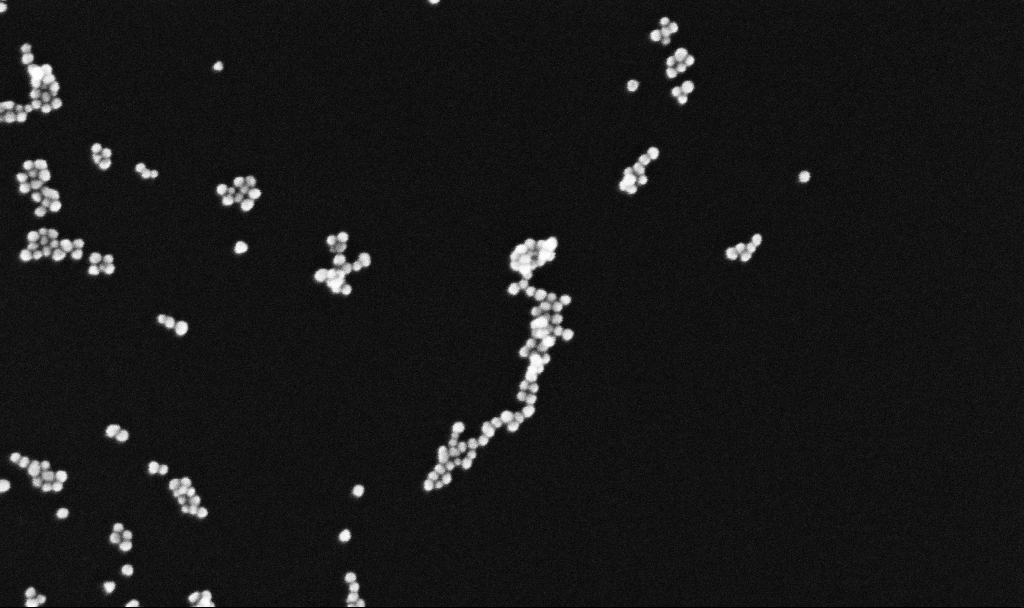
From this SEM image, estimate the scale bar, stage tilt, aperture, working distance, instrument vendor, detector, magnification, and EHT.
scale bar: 100 nm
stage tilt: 0°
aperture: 30 µm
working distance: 3.4 mm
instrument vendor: Zeiss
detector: InLens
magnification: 300 K X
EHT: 10 kV